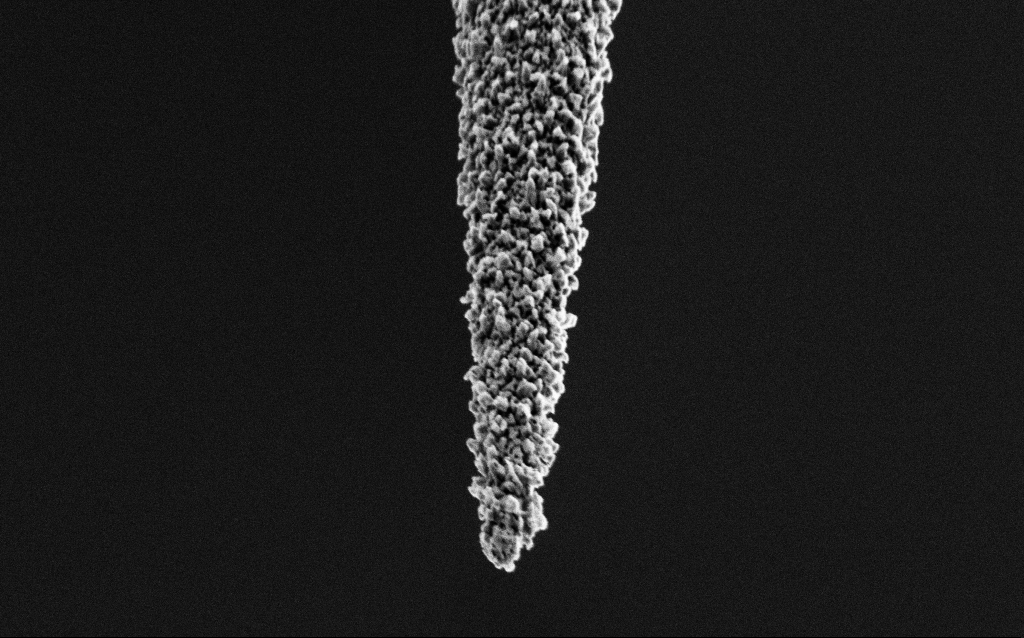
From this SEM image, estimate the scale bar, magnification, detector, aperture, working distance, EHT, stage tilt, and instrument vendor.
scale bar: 1000 nm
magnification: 40 K X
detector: SE2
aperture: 30 µm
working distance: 6.4 mm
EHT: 2 kV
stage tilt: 44.9°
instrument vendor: Zeiss